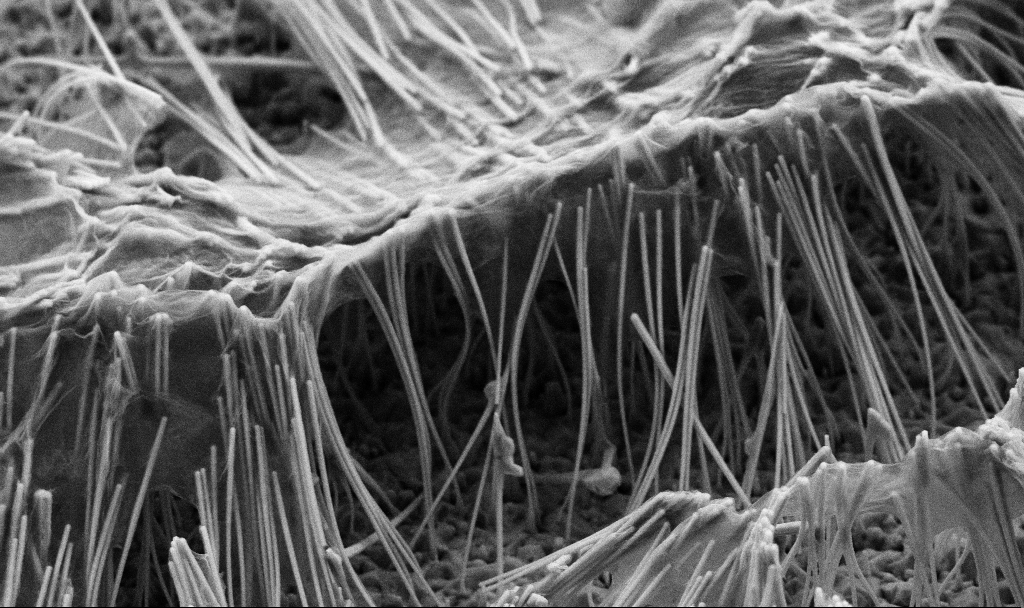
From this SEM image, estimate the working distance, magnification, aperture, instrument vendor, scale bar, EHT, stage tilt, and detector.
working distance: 7.2 mm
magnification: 17.41 K X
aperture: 30 µm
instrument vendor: Zeiss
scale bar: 2000 nm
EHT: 5 kV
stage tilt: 45°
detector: SE2